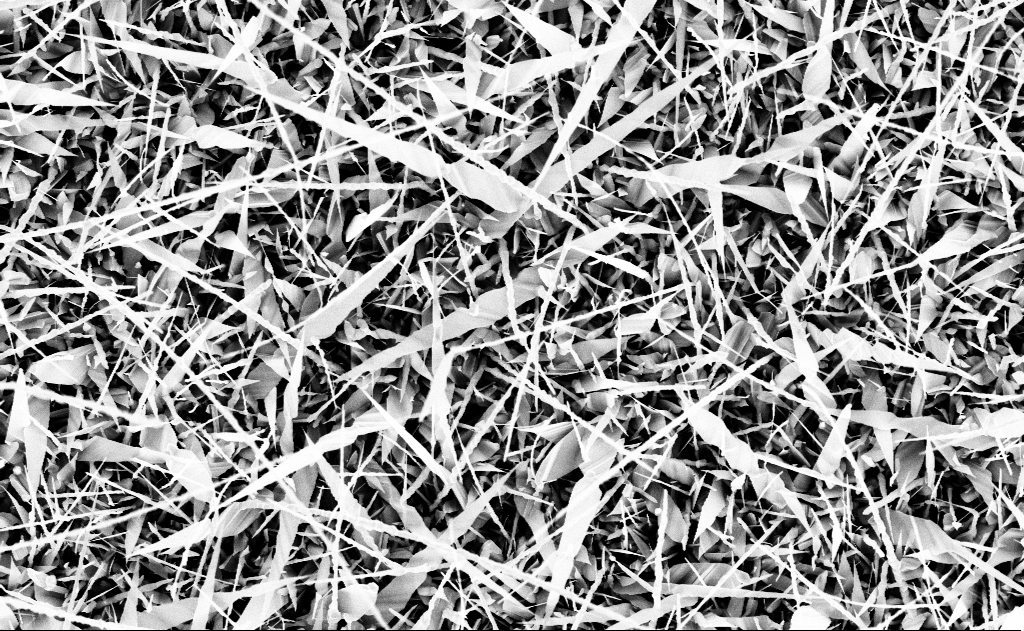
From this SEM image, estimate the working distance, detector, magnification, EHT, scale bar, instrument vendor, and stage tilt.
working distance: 13 mm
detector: InLens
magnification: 10 K X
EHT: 10 kV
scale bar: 2000 nm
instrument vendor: Zeiss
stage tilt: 0°